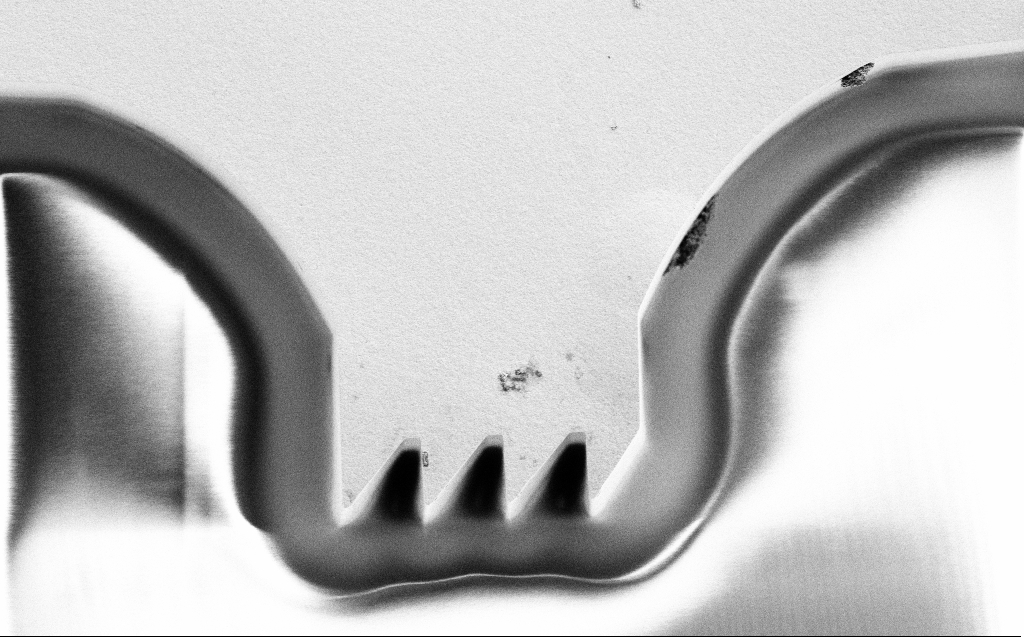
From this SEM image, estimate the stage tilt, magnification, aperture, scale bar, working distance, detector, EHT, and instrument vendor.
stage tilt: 0°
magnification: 2.63 K X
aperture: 30 µm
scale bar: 10000 nm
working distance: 9 mm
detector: SE2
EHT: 2 kV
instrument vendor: Zeiss